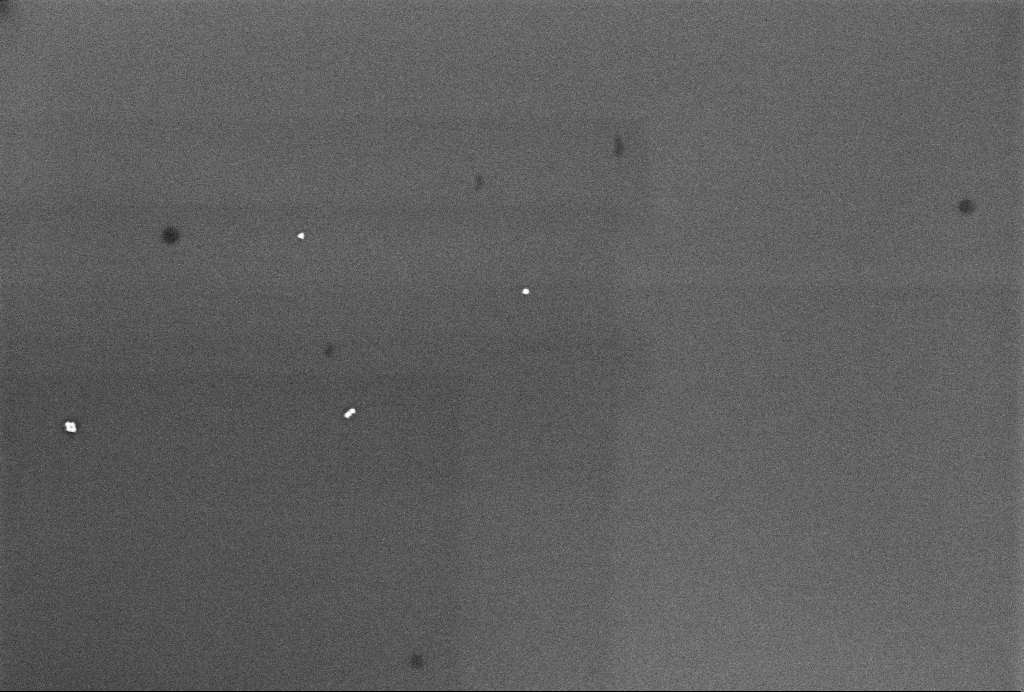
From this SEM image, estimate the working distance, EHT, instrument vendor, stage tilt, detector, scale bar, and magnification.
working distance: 3.3 mm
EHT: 2 kV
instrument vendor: Zeiss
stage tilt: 0°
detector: InLens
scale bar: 200 nm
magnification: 74.02 K X